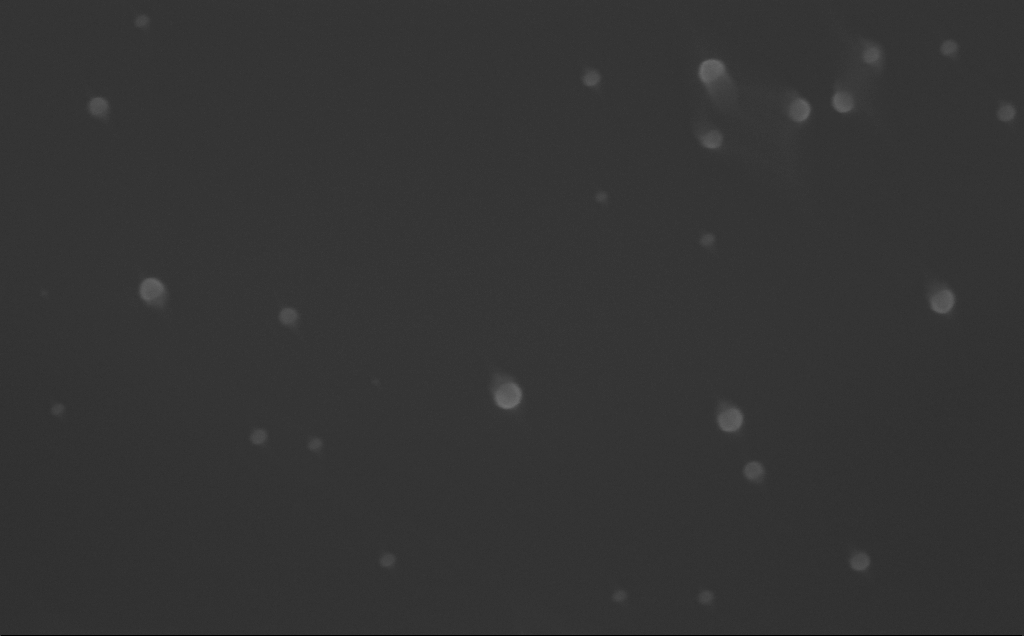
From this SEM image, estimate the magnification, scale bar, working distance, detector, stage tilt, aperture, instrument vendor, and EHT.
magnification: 150 K X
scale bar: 100 nm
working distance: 6 mm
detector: InLens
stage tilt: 0°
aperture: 30 µm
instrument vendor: Zeiss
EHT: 10 kV